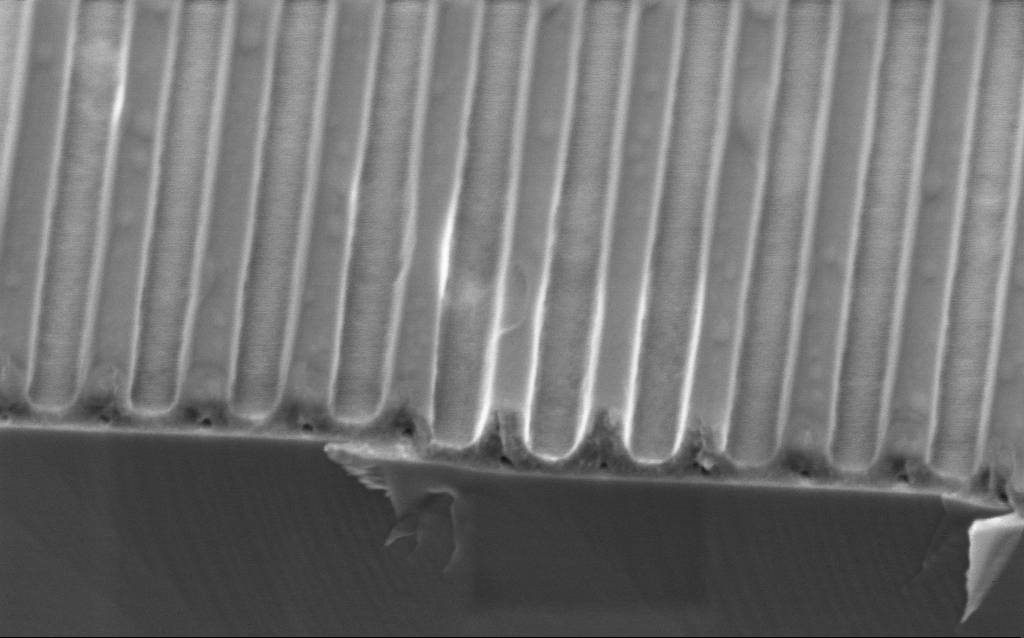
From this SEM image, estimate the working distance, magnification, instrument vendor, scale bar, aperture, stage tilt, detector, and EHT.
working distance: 3.8 mm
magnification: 73.82 K X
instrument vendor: Zeiss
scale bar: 200 nm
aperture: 30 µm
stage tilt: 45°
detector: InLens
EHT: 2 kV